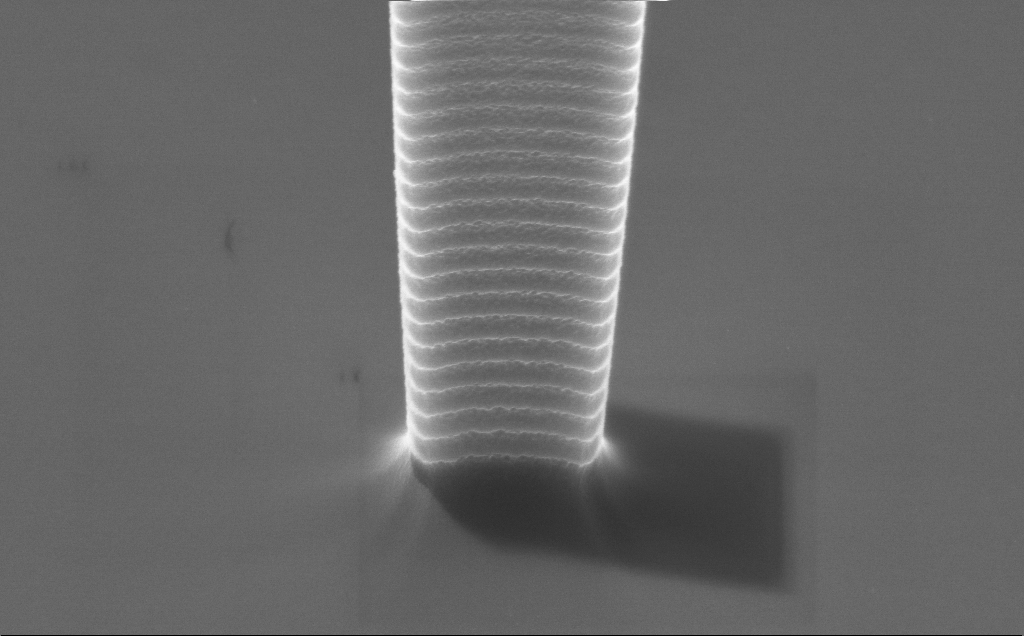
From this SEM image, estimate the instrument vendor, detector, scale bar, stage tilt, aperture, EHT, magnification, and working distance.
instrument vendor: Zeiss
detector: InLens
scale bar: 2000 nm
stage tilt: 45°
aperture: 30 µm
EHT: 10 kV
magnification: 22.6 K X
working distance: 4 mm